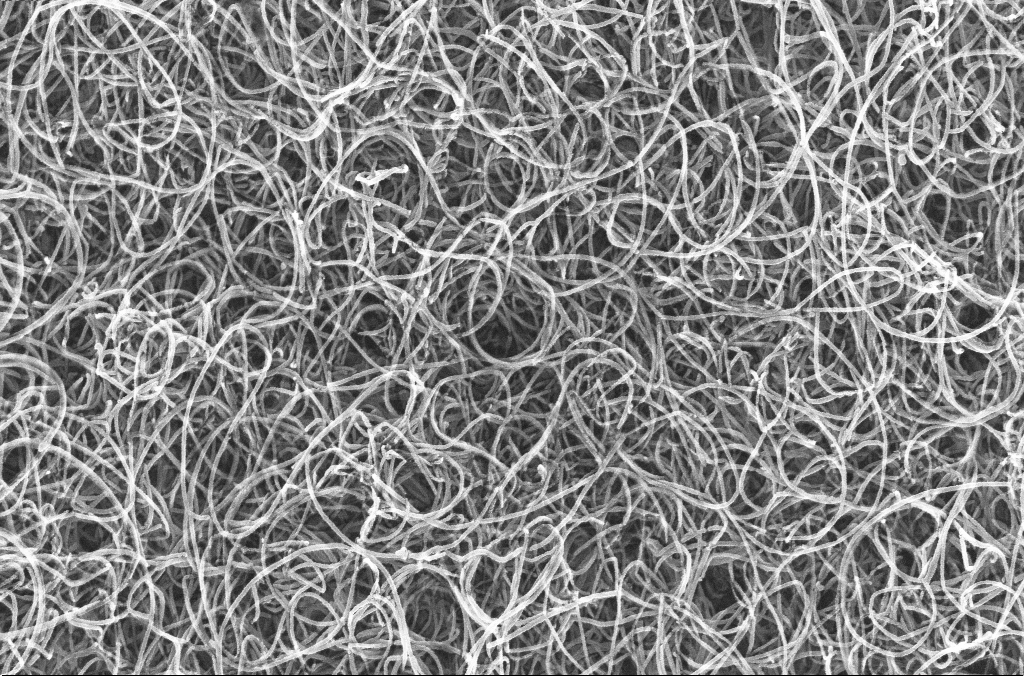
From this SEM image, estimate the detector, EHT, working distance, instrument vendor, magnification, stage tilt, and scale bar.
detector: InLens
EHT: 20 kV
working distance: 4.5 mm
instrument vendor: Zeiss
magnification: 50 K X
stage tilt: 0°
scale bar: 1000 nm